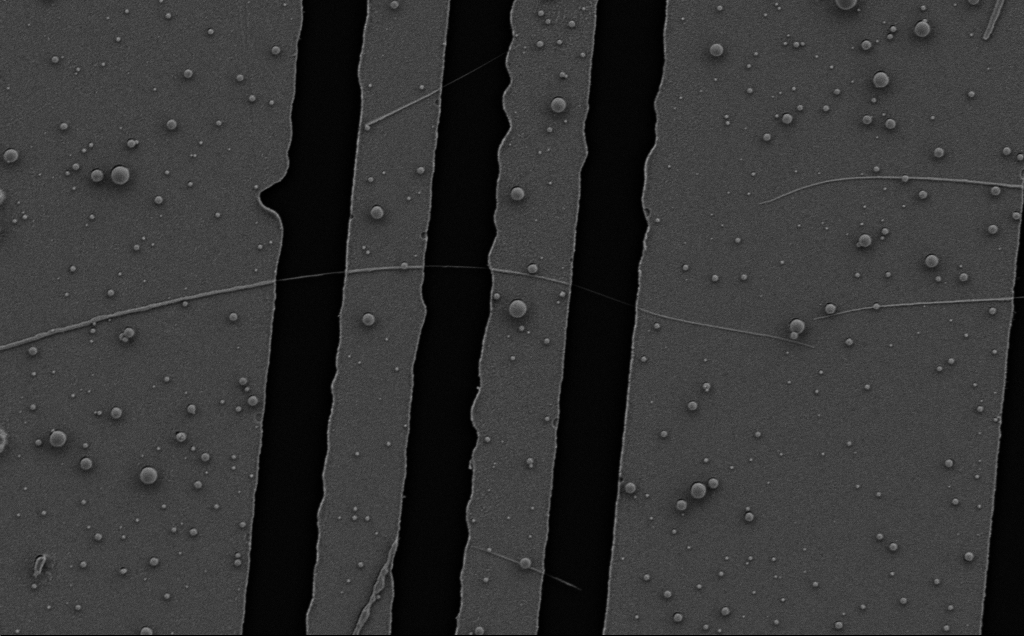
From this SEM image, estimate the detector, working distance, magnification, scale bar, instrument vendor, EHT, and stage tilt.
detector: SE2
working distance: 8 mm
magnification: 13.58 K X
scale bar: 2000 nm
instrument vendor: Zeiss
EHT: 5 kV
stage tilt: -0.7°